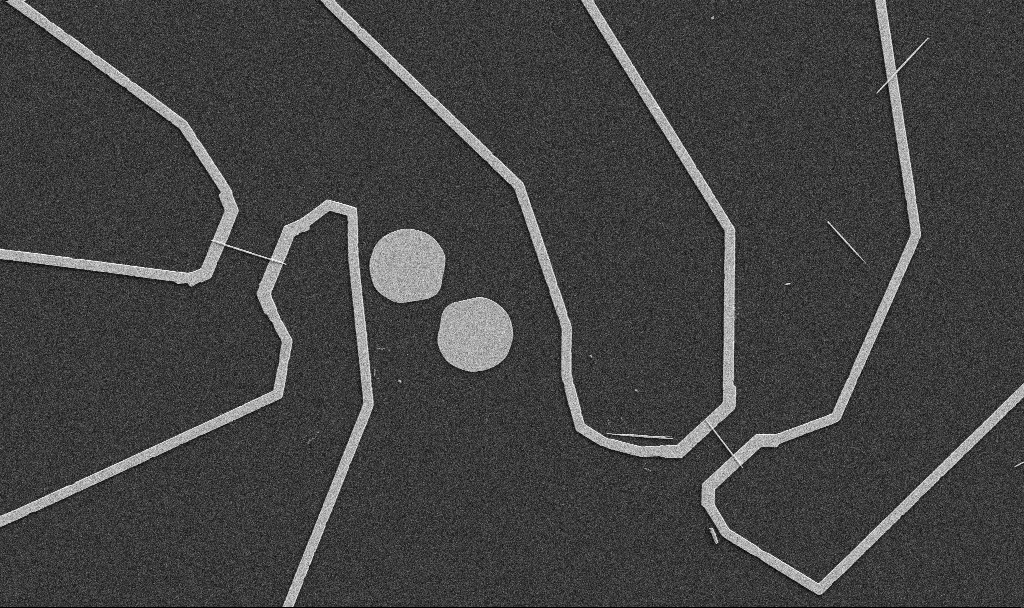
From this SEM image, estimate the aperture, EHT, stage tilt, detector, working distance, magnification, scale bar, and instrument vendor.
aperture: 30 µm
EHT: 5 kV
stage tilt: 0°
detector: SE2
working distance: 10.7 mm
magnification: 5 K X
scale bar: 10000 nm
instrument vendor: Zeiss